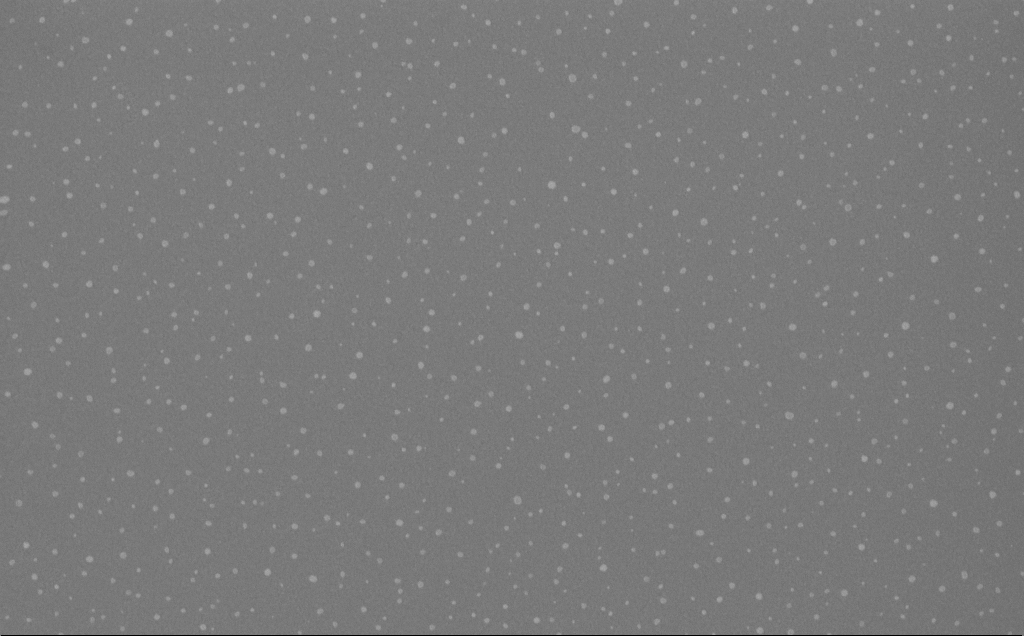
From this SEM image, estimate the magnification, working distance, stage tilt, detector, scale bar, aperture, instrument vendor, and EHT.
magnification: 40 K X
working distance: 5 mm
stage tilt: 0°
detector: InLens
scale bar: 1000 nm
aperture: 30 µm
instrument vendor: Zeiss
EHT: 10 kV